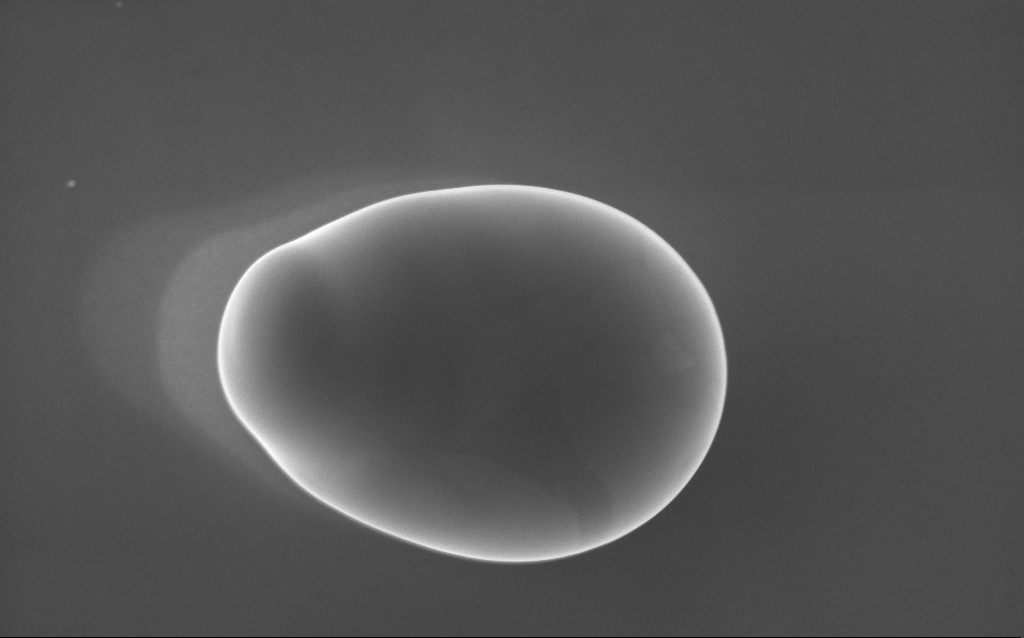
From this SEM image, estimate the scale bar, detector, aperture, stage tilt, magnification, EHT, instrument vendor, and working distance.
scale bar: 1000 nm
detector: InLens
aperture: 30 µm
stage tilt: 0°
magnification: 58.59 K X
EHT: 10 kV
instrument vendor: Zeiss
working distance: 3 mm